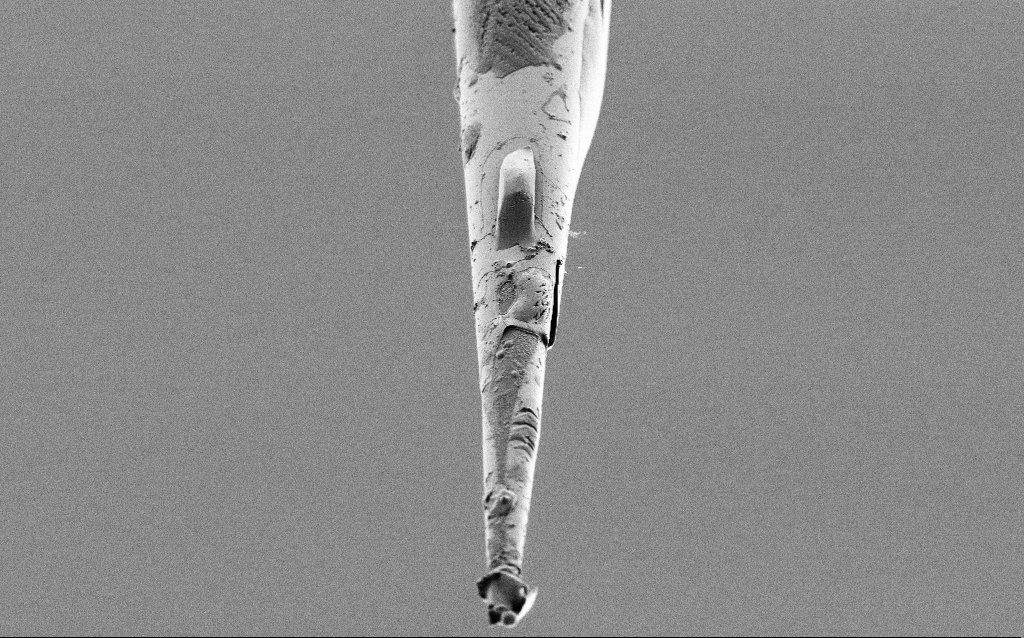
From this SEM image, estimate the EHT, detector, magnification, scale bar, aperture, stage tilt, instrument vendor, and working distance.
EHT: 1 kV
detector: SE2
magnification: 5 K X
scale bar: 10000 nm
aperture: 30 µm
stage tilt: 45°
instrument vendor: Zeiss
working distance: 6.5 mm